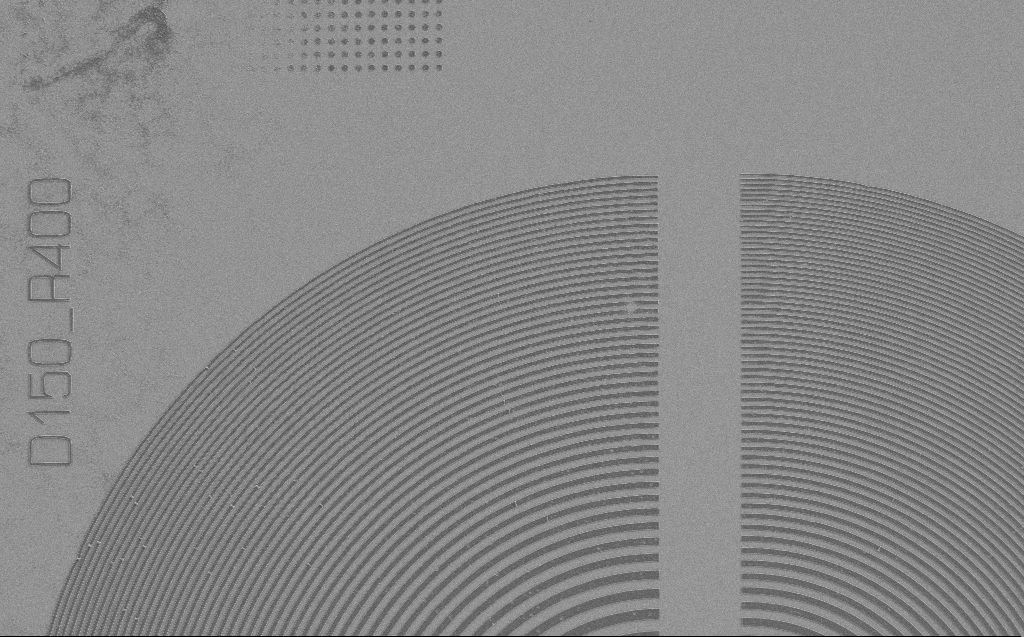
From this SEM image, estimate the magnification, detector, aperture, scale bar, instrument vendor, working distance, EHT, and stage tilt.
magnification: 3.1 K X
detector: SE2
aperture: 30 µm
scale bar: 20000 nm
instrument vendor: Zeiss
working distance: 5 mm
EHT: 1.2 kV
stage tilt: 0°